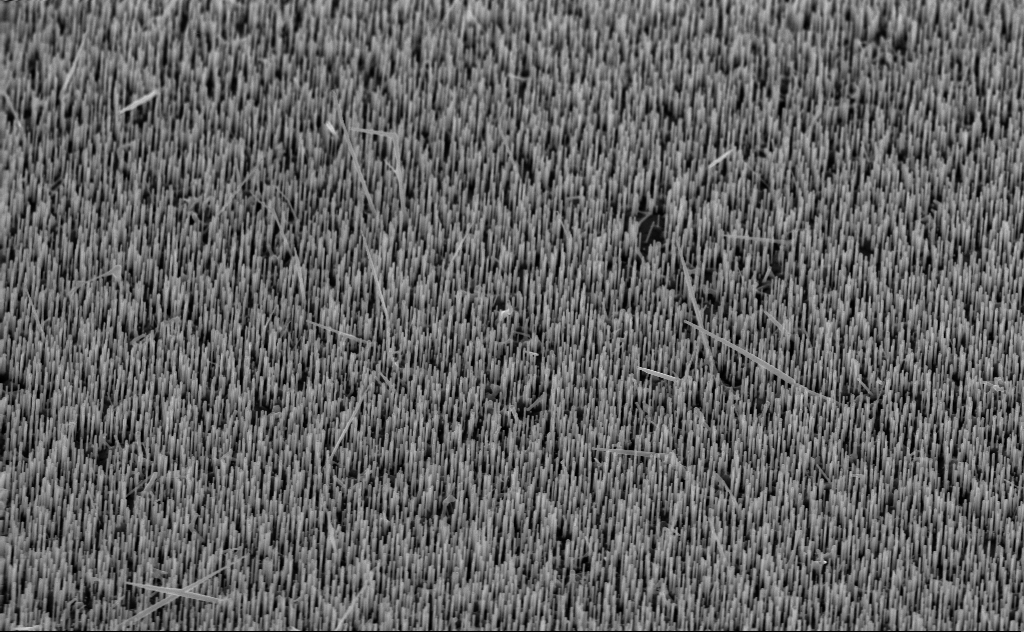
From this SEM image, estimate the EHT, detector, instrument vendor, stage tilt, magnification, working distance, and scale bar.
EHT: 10 kV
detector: InLens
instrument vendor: Zeiss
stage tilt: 45°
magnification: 20 K X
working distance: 6 mm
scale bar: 1000 nm